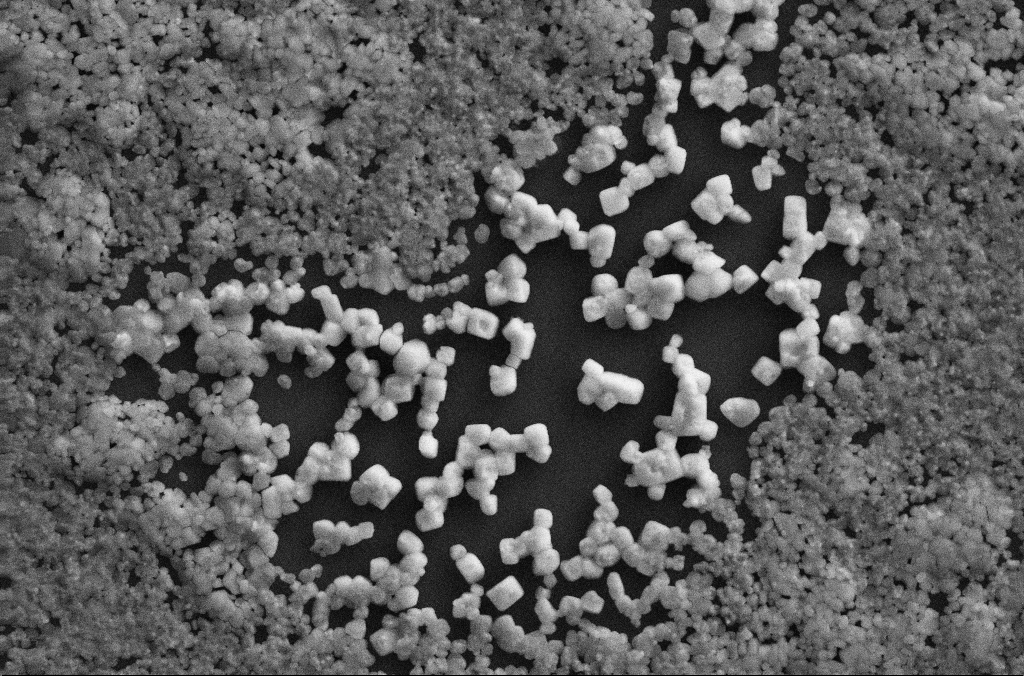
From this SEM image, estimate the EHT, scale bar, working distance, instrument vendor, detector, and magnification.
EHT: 20 kV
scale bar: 10000 nm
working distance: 2.8 mm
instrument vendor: Zeiss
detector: SE2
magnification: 4 K X